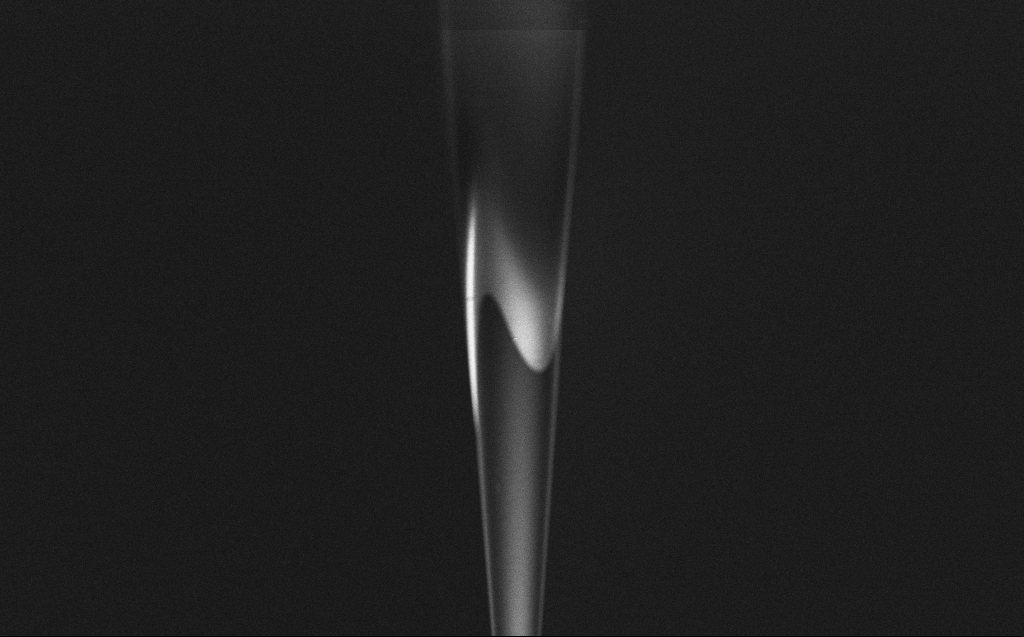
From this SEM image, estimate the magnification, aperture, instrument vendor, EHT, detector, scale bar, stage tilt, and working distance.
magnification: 5 K X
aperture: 30 µm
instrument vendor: Zeiss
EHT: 2 kV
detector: InLens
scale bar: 10000 nm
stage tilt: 45°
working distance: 6 mm